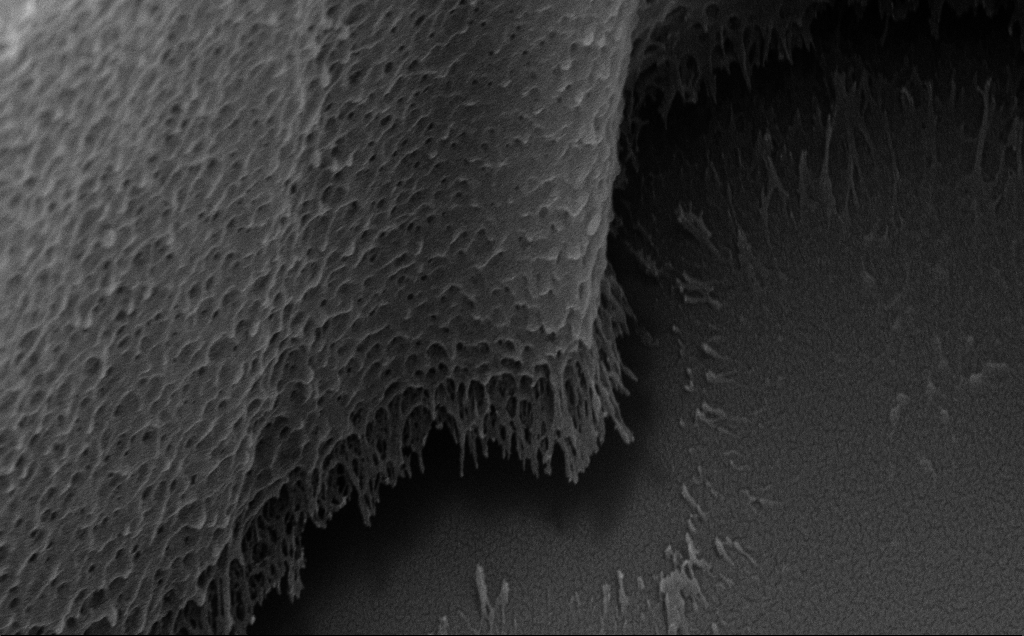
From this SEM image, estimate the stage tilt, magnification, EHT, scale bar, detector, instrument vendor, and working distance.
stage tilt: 35.3°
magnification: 41.18 K X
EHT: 10 kV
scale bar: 1000 nm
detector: SE2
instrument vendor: Zeiss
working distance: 11 mm